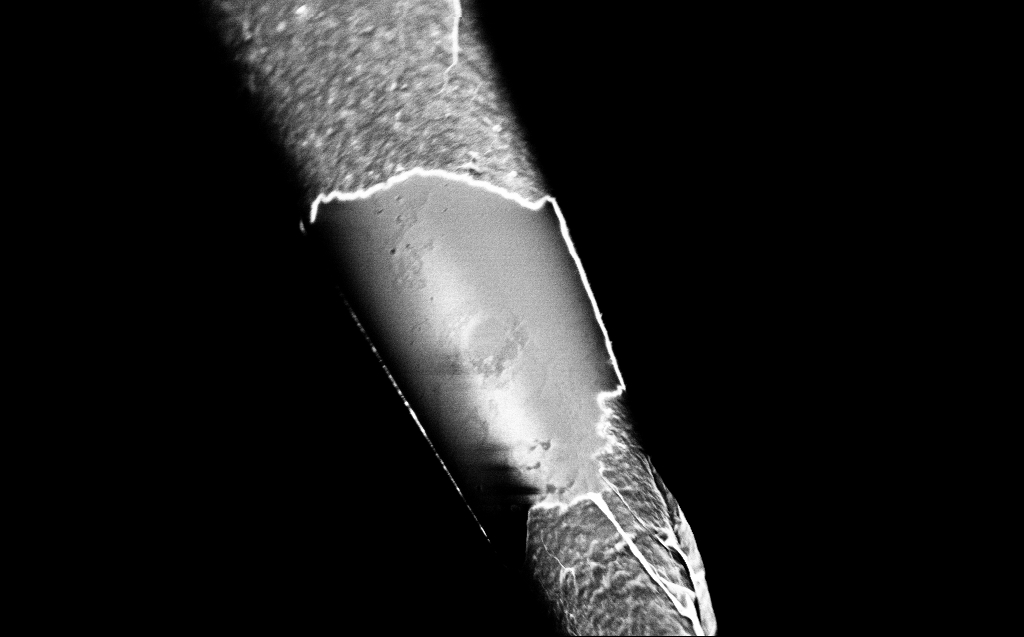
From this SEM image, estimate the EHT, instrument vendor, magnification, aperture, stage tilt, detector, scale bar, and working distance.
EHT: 2 kV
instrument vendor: Zeiss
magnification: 25 K X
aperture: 30 µm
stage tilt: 45°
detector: InLens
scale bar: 2000 nm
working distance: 3 mm